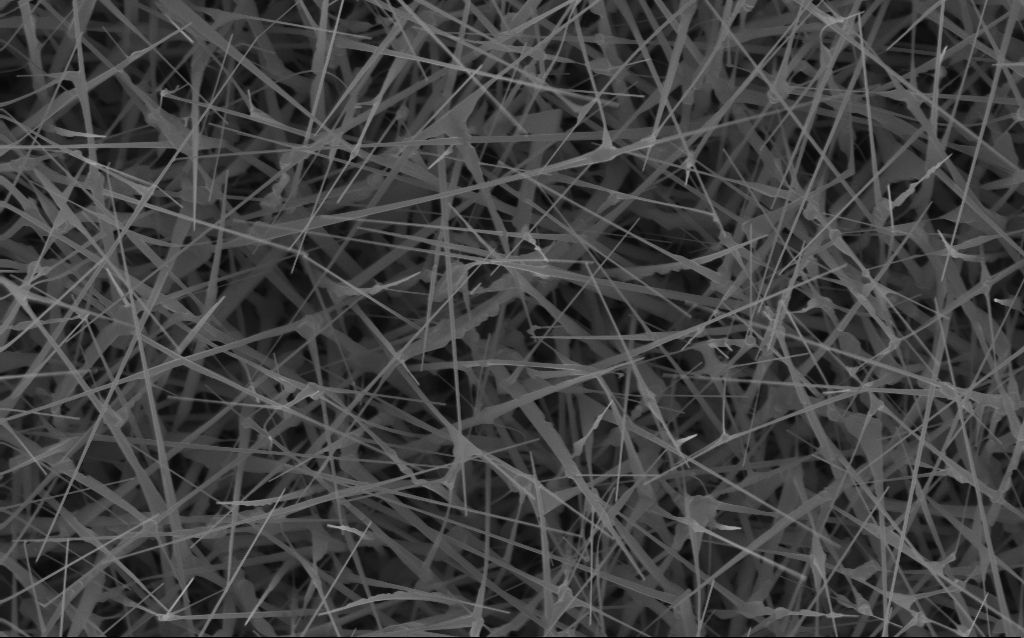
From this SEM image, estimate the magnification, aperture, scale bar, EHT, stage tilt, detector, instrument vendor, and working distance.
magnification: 20 K X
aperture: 30 µm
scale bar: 2000 nm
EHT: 10 kV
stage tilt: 0°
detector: InLens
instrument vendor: Zeiss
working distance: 6 mm